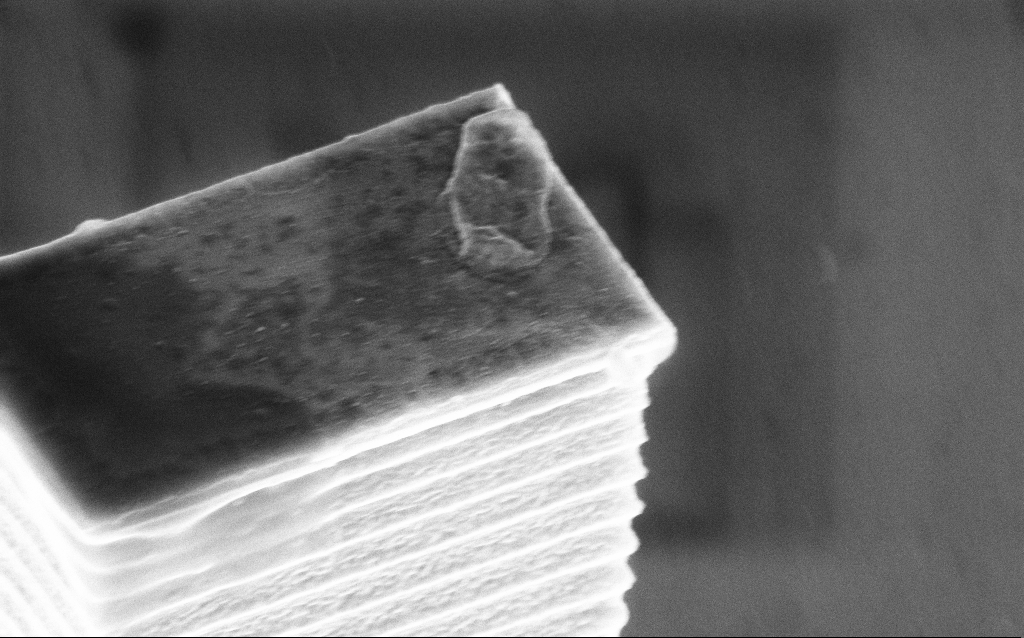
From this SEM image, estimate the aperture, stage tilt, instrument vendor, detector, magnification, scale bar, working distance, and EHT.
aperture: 30 µm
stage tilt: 45°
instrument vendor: Zeiss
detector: InLens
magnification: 50.07 K X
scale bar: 1000 nm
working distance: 4 mm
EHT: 5 kV